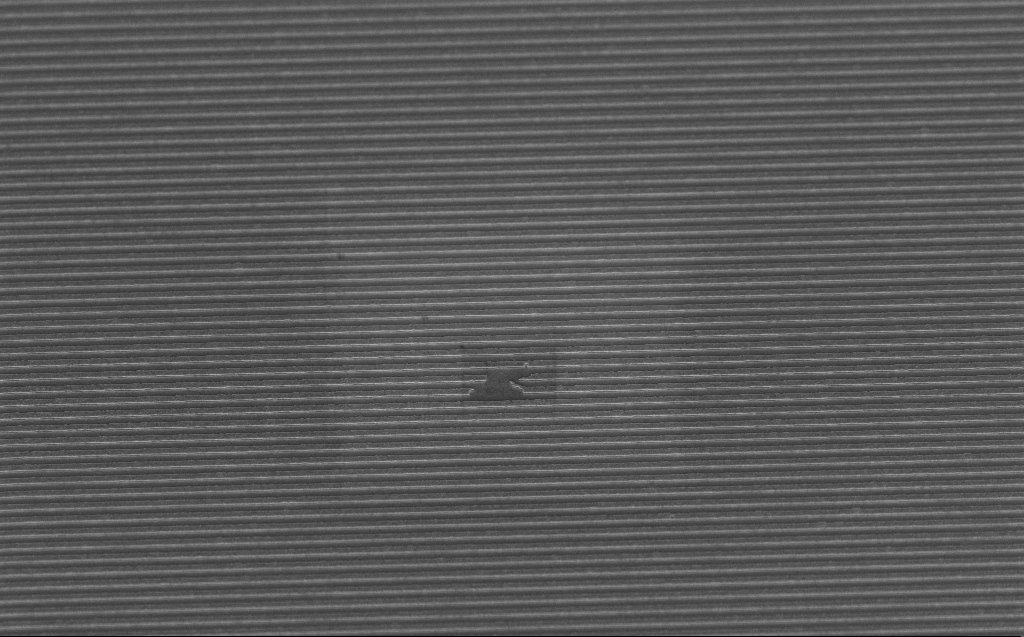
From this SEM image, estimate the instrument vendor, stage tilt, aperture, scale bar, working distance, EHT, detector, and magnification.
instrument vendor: Zeiss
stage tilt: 45°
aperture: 30 µm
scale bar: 2000 nm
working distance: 5 mm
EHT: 5 kV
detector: InLens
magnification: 9.97 K X